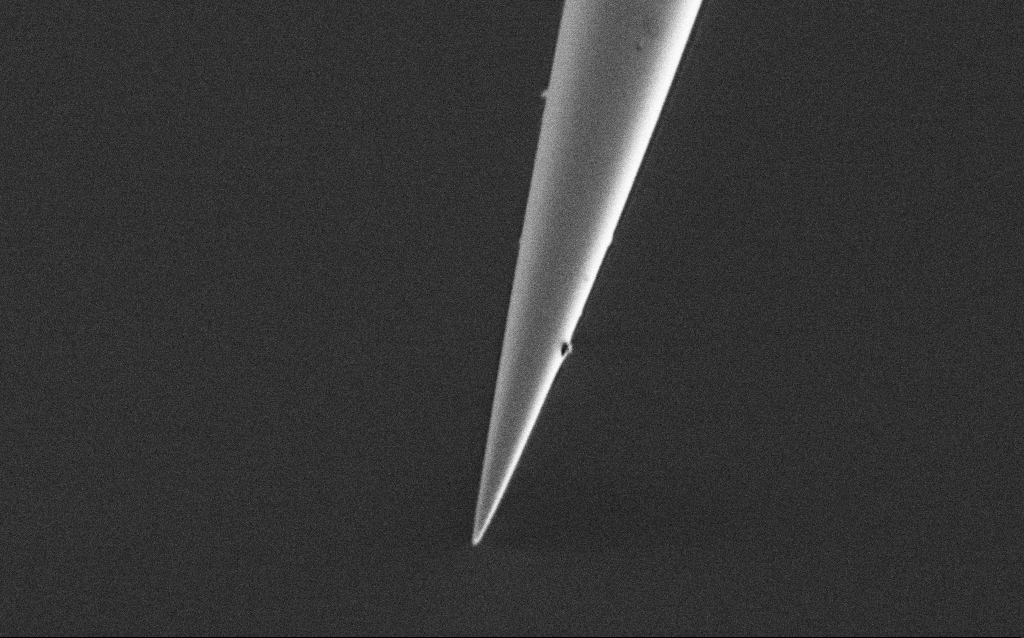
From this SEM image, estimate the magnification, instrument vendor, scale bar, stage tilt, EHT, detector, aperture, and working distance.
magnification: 25 K X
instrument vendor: Zeiss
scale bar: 2000 nm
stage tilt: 45°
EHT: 1 kV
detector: SE2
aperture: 30 µm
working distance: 6.6 mm